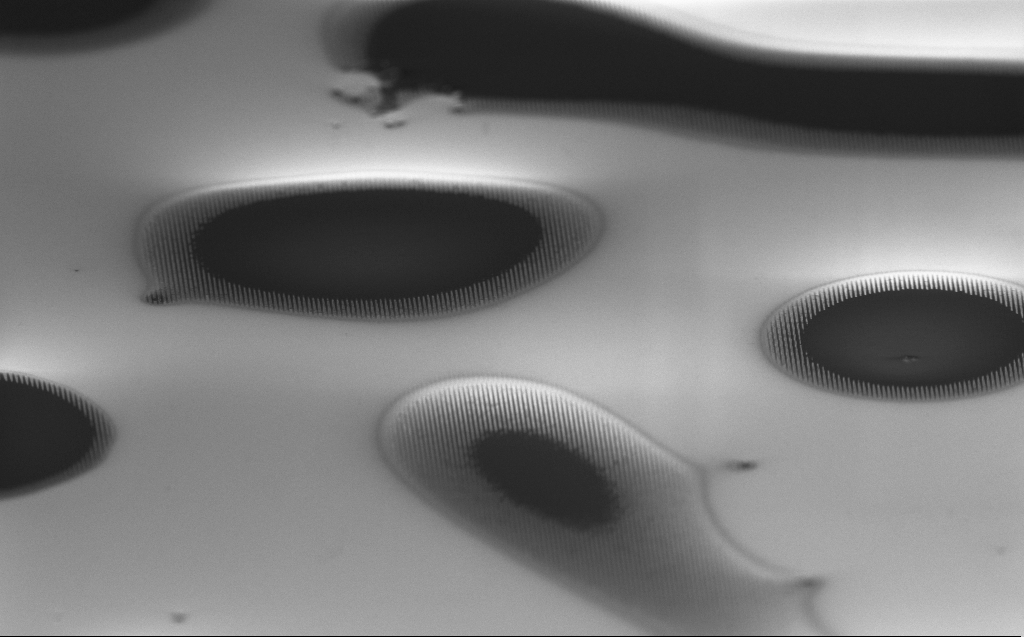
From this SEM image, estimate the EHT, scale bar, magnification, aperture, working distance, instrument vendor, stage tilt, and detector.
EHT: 1 kV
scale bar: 10000 nm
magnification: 3.59 K X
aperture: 30 µm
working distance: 3 mm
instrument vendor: Zeiss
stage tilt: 60.7°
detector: InLens